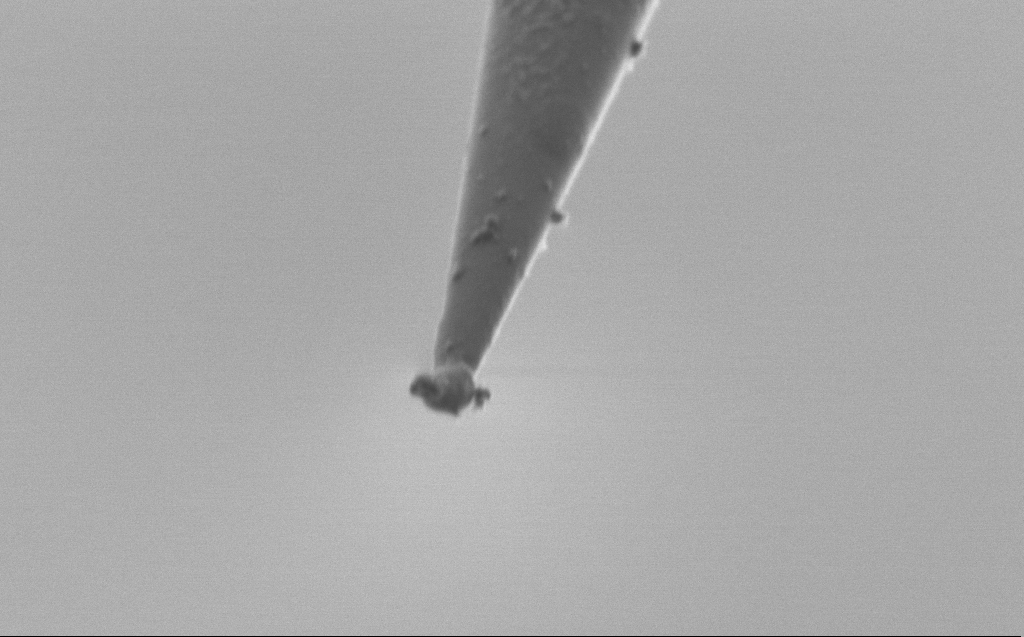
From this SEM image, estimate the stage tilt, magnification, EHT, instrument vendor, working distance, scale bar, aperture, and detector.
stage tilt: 45°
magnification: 50 K X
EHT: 1 kV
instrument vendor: Zeiss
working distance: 5 mm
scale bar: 1000 nm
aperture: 30 µm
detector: SE2